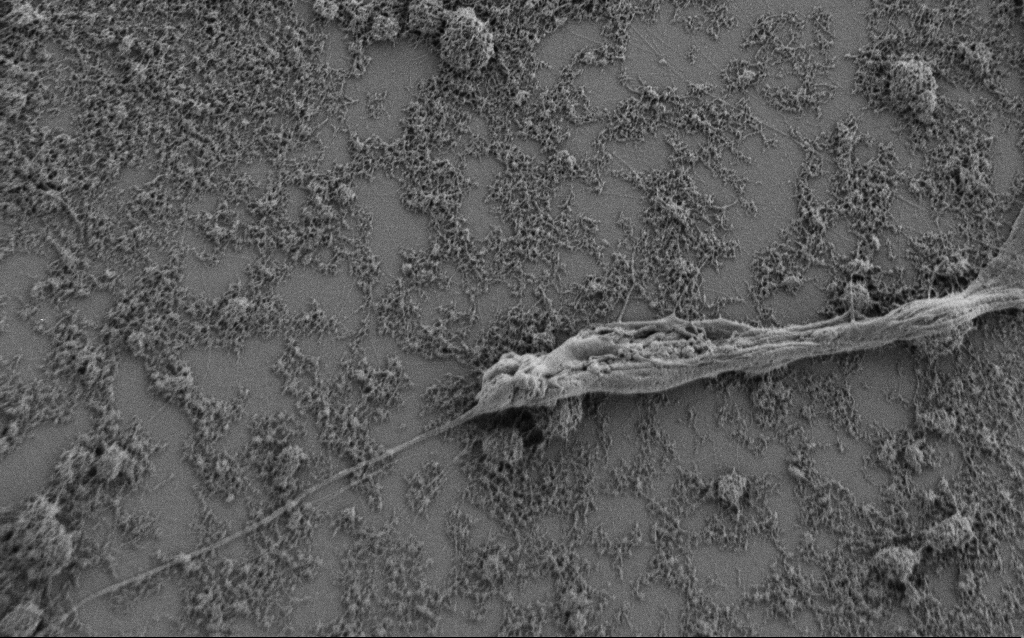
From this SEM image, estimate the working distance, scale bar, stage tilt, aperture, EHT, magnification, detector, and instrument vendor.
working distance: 7 mm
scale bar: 2000 nm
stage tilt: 0°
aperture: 30 µm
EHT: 0.9 kV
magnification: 7.5 K X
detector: SE2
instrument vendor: Zeiss